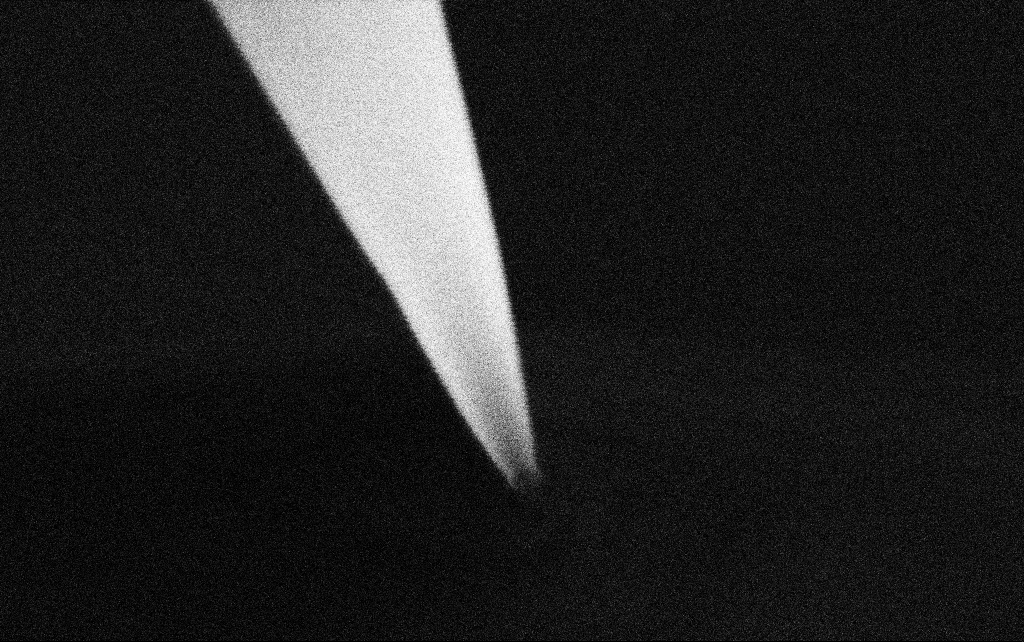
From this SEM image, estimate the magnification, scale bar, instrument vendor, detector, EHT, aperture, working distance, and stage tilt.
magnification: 200 K X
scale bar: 200 nm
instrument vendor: Zeiss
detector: SE2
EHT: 3 kV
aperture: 30 µm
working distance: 7.7 mm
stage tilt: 45°